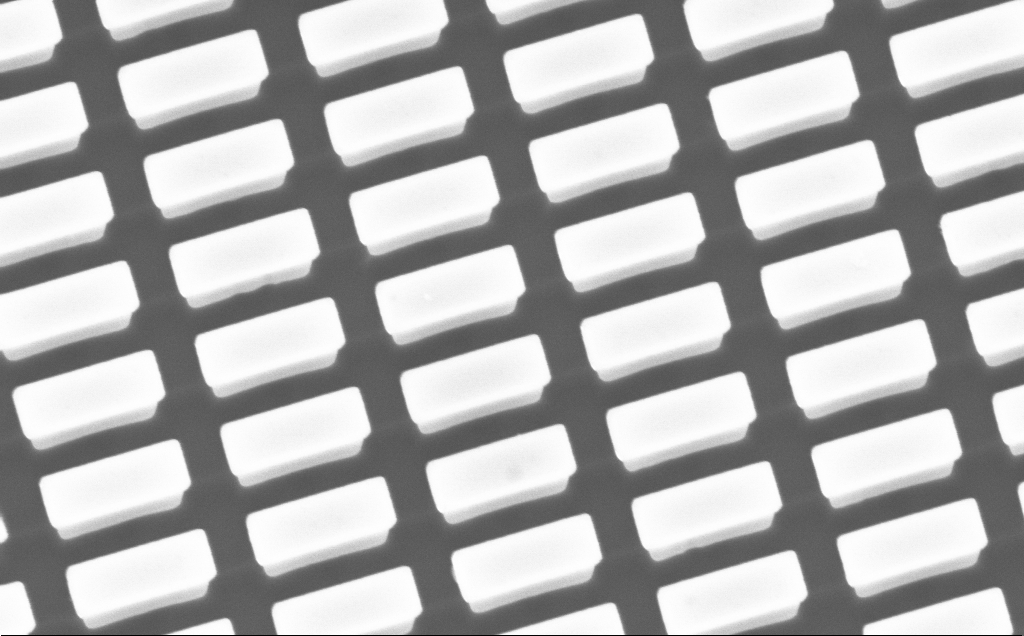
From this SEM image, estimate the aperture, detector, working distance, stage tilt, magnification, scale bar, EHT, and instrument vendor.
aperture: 30 µm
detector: InLens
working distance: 6 mm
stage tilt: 3.3°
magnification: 17.31 K X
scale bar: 1000 nm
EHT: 10 kV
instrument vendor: Zeiss